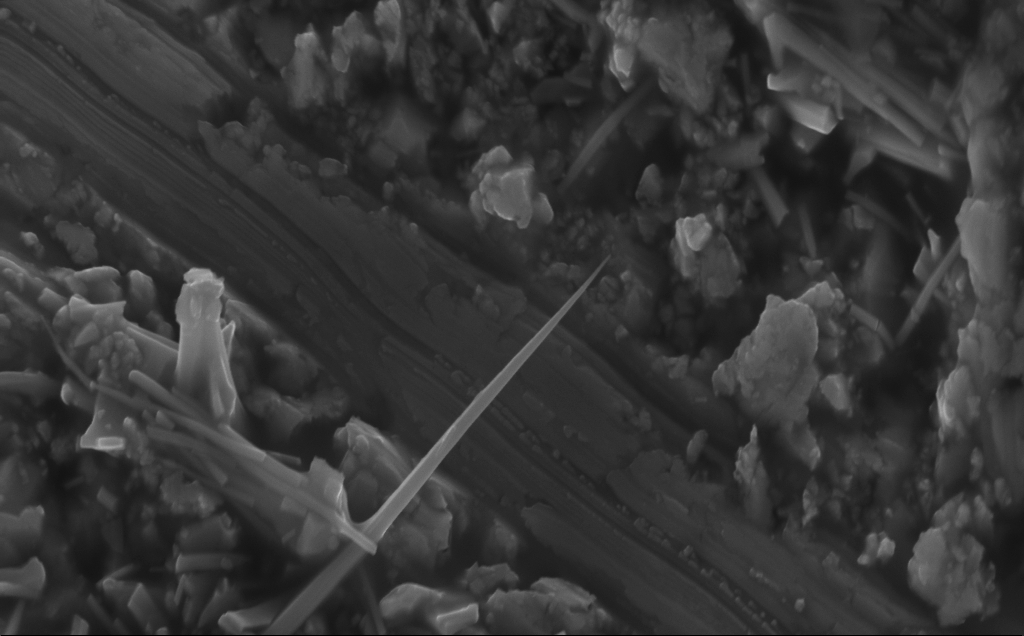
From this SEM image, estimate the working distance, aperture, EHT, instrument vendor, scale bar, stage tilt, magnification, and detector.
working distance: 7 mm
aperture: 30 µm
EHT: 10 kV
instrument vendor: Zeiss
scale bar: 1000 nm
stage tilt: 30°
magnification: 36.72 K X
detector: InLens